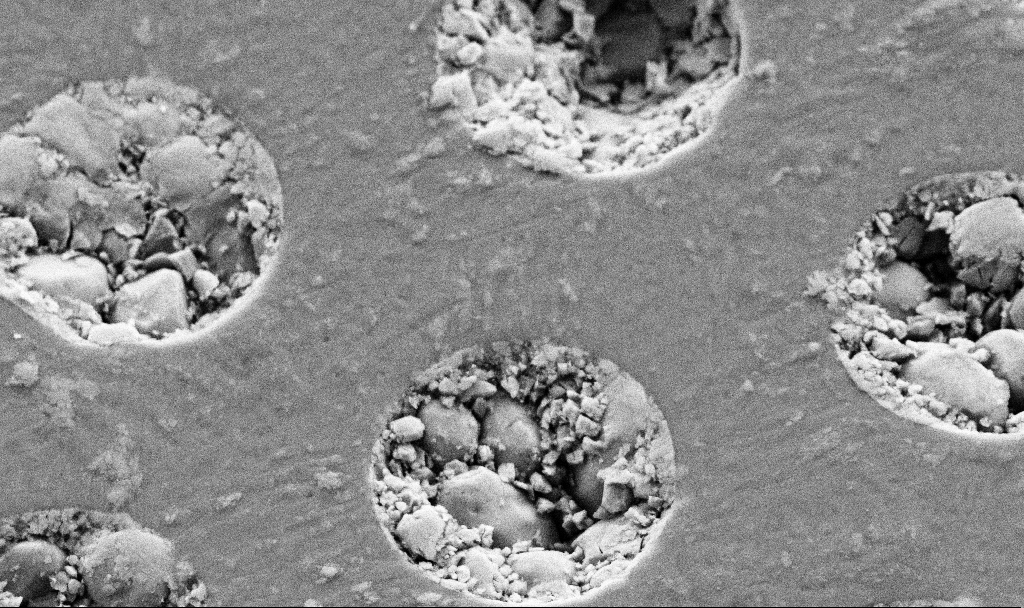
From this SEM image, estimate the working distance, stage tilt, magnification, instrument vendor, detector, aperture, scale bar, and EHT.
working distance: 4.5 mm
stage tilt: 30°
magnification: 30.4 K X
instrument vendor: Zeiss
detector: SE2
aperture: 30 µm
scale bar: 1000 nm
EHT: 3 kV